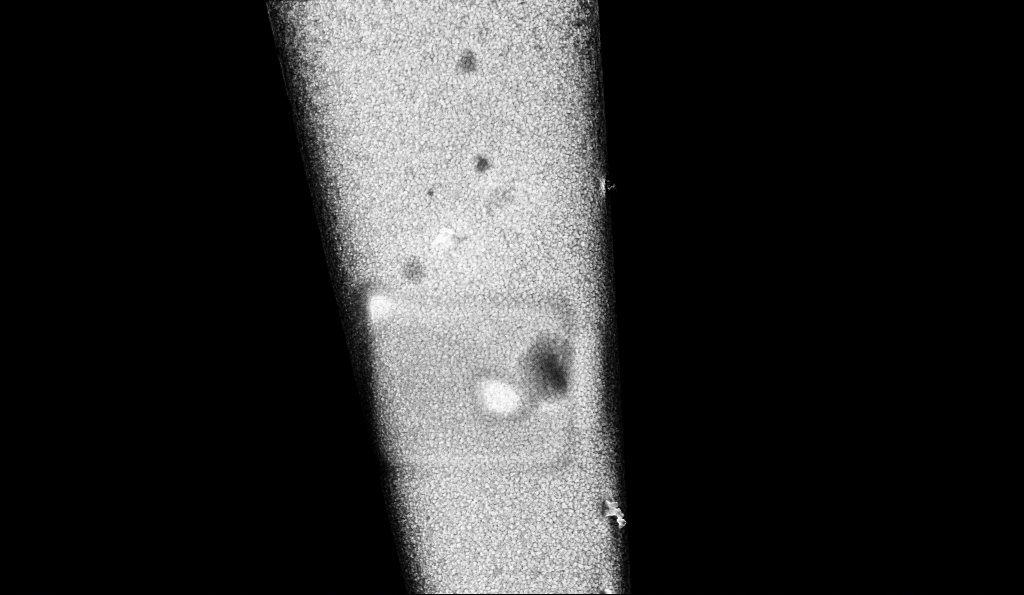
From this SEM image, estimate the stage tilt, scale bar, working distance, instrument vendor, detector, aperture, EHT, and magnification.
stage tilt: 1.3°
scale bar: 1000 nm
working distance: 3.8 mm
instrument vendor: Zeiss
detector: InLens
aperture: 30 µm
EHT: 10 kV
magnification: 28.03 K X